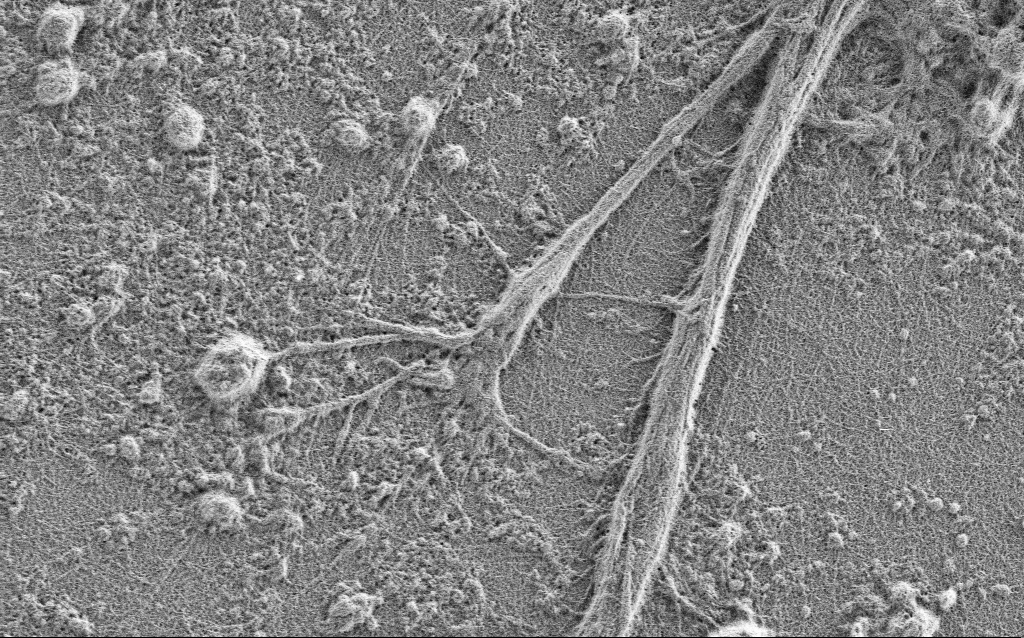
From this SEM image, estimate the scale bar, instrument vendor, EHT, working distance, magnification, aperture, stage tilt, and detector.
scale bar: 2000 nm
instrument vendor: Zeiss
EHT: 0.9 kV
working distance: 4 mm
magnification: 7.5 K X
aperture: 30 µm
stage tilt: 0°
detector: SE2